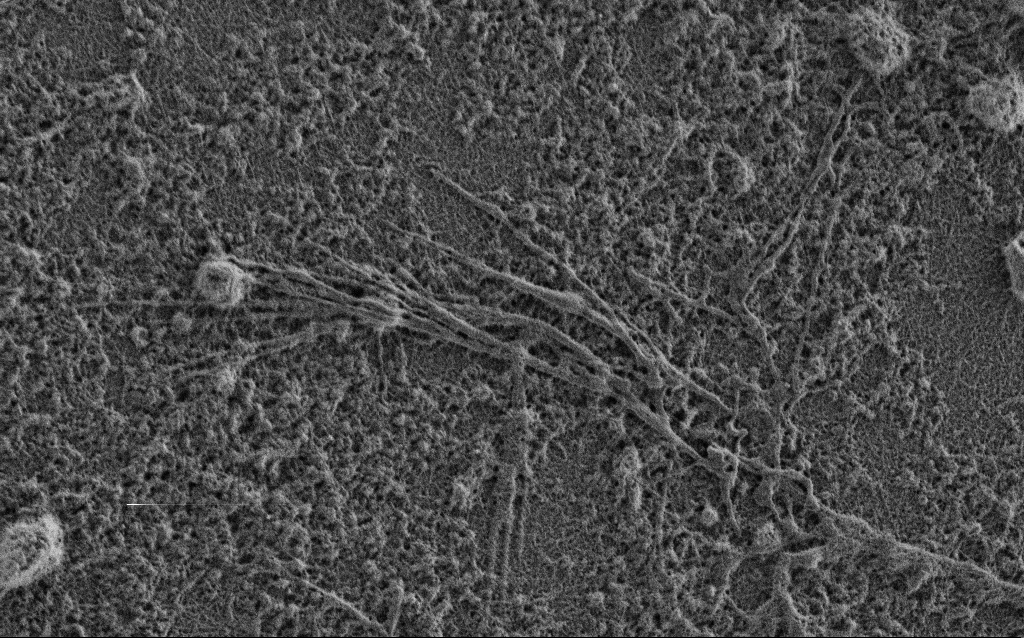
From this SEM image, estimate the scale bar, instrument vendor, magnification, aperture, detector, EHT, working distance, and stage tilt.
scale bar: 2000 nm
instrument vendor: Zeiss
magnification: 15 K X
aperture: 30 µm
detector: SE2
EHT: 0.9 kV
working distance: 3.4 mm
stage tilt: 0°